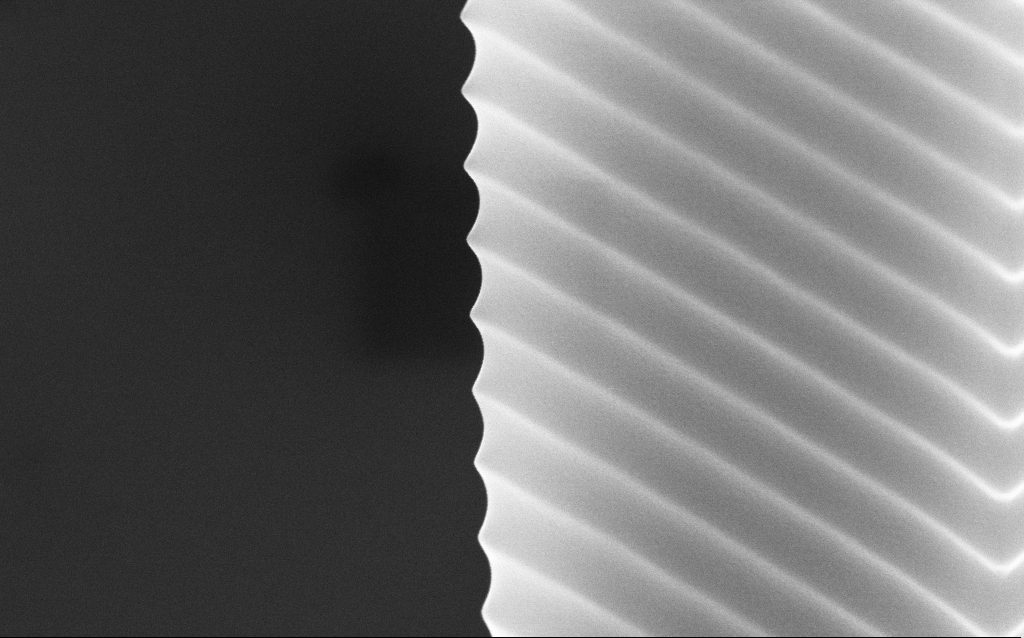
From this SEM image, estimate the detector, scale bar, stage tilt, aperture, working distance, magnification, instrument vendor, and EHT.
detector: InLens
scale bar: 200 nm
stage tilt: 45°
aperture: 30 µm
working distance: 5.1 mm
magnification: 109.95 K X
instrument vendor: Zeiss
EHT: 10 kV